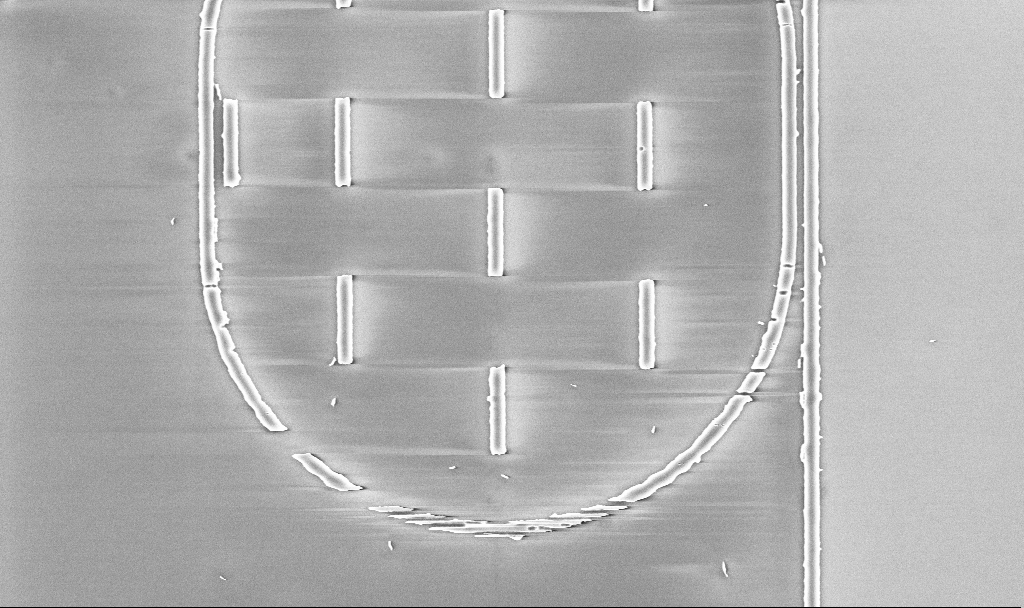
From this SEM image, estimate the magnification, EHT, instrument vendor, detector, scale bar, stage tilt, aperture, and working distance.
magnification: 11.07 K X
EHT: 5 kV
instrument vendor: Zeiss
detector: InLens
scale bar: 2000 nm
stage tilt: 0°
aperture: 30 µm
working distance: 5.2 mm